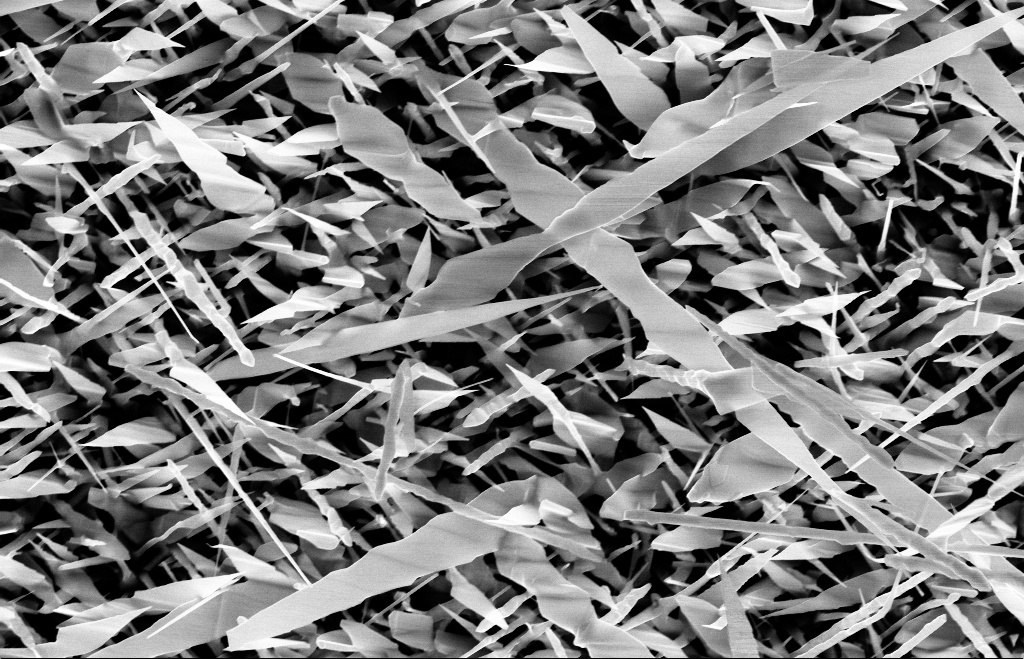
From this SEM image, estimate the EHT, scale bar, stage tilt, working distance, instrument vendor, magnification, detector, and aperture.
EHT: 10 kV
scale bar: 1000 nm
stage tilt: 0°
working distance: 8 mm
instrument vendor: Zeiss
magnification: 20 K X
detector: InLens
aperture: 30 µm